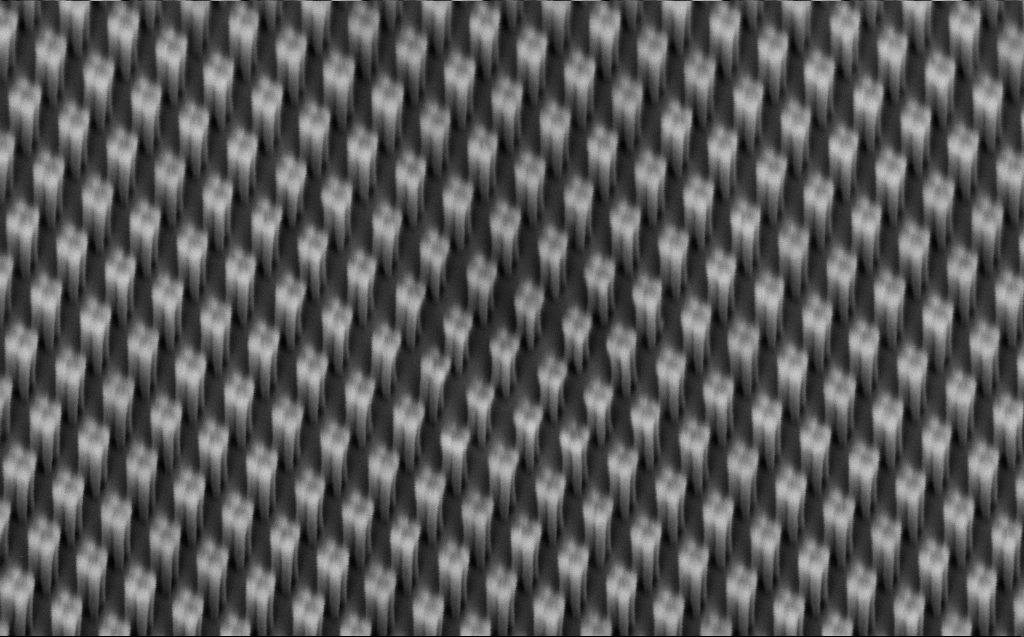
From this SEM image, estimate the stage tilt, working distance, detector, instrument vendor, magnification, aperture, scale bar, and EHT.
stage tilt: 42.8°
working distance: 6 mm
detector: InLens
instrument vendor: Zeiss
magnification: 40 K X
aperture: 30 µm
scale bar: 1000 nm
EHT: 1.2 kV